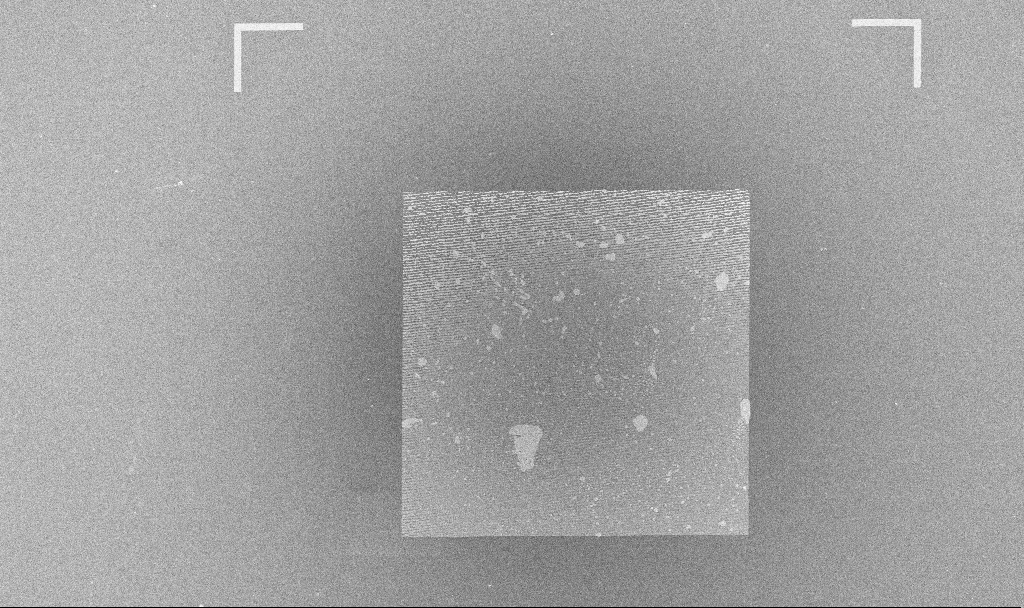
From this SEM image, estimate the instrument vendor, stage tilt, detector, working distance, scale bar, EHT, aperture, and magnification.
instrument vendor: Zeiss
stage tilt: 0°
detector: InLens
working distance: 3.6 mm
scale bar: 100000 nm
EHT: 5 kV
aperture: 30 µm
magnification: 0.253 K X